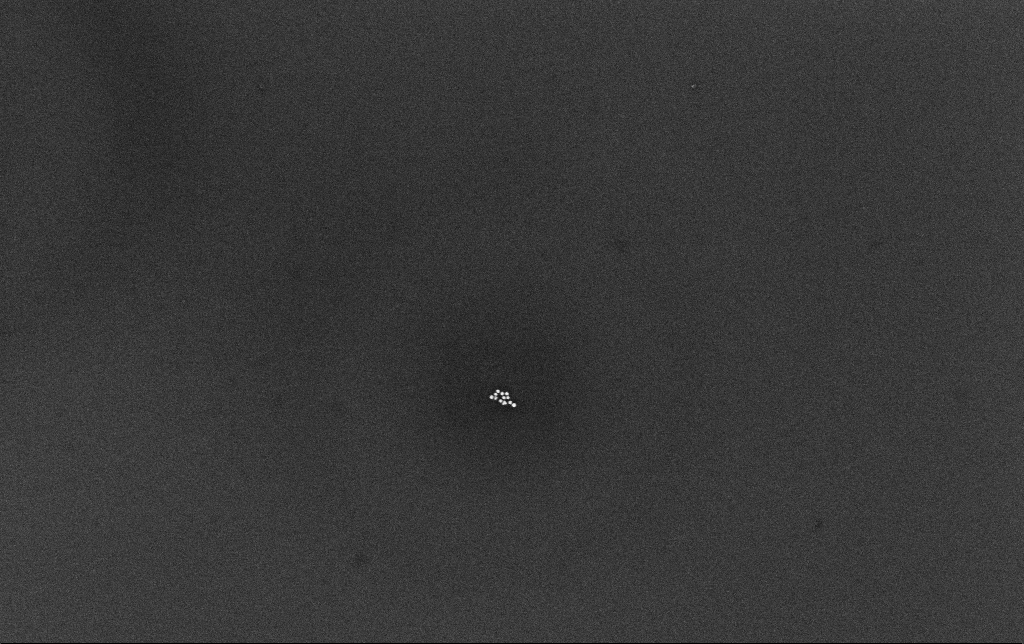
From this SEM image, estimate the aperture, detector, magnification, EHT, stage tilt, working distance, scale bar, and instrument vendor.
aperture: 30 µm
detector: InLens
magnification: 100 K X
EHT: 10 kV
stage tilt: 0°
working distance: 3.4 mm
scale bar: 200 nm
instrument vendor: Zeiss